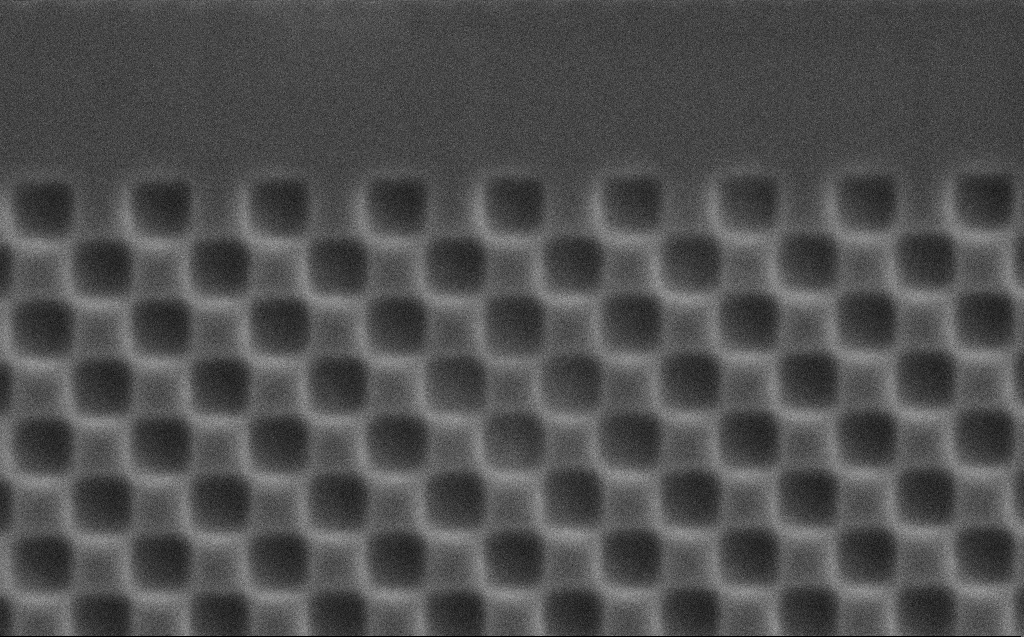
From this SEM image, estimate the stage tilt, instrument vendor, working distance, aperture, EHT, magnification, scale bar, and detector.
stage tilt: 0°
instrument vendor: Zeiss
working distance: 3 mm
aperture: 30 µm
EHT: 5 kV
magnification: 21.84 K X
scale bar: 1000 nm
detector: SE2